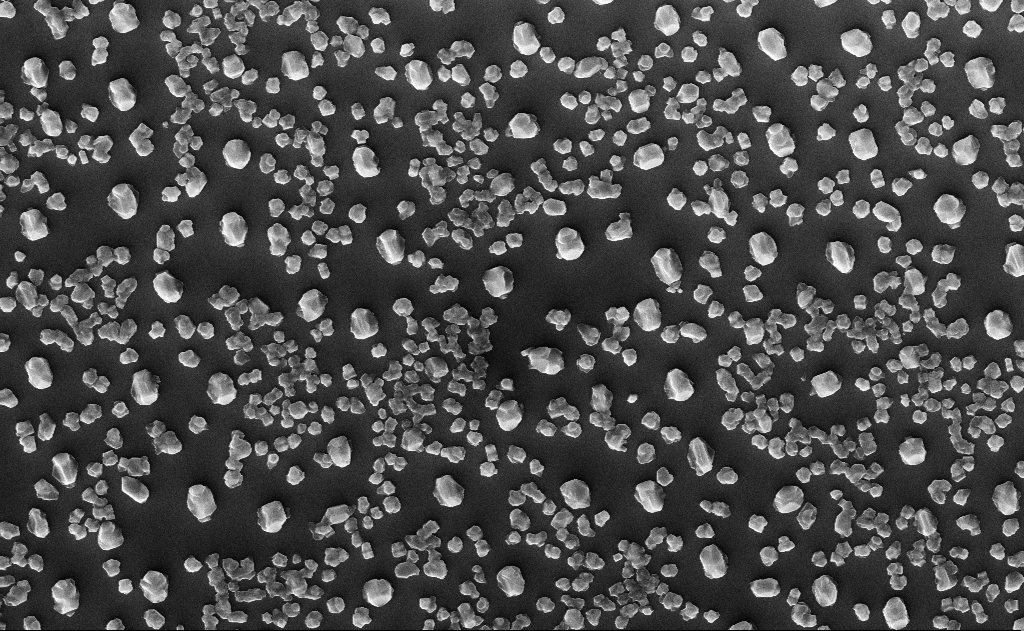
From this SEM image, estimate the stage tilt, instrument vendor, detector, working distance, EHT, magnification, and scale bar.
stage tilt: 0°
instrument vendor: Zeiss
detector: InLens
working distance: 18 mm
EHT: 10 kV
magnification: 20 K X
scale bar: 1000 nm